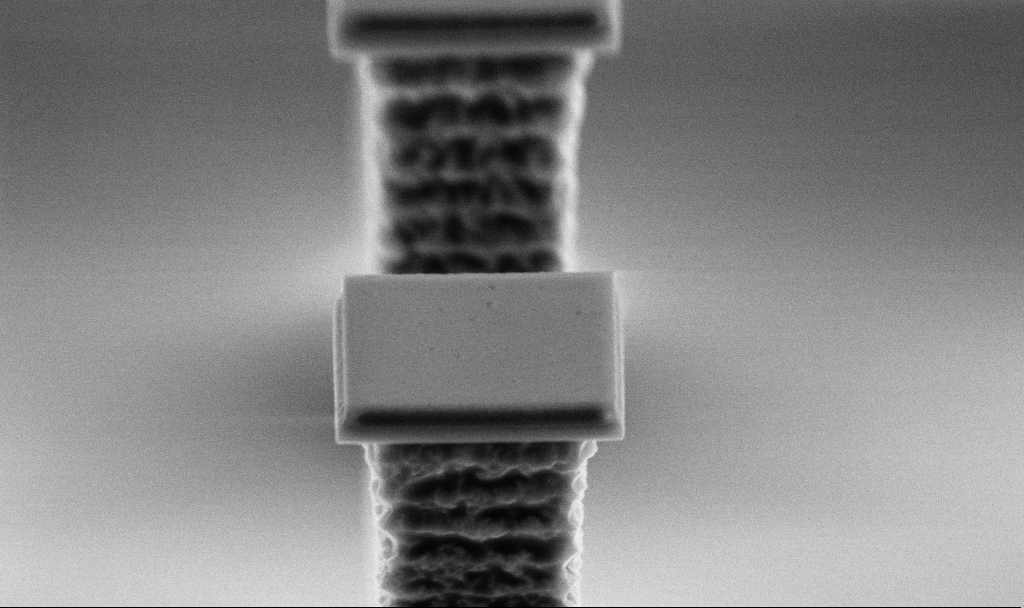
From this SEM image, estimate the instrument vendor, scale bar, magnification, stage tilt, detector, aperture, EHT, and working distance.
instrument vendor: Zeiss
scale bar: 1000 nm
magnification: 36.71 K X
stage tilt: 70°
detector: SE2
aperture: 30 µm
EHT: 5 kV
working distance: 5.7 mm